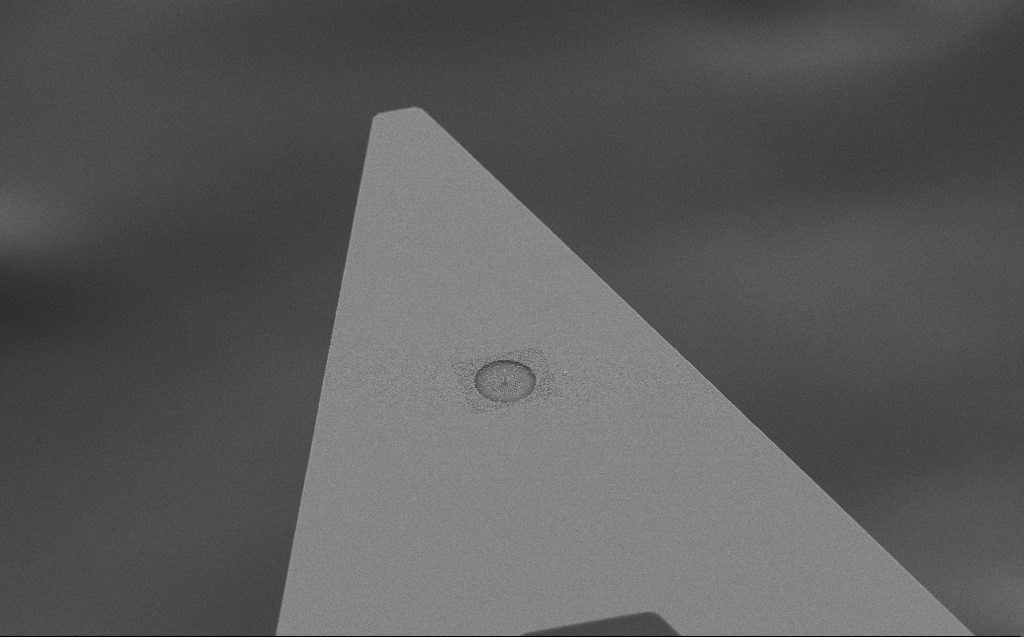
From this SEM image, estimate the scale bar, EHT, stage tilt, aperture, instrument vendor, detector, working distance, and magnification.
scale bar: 10000 nm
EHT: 10 kV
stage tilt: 45°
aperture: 30 µm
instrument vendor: Zeiss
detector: SE2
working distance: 6 mm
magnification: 3.2 K X